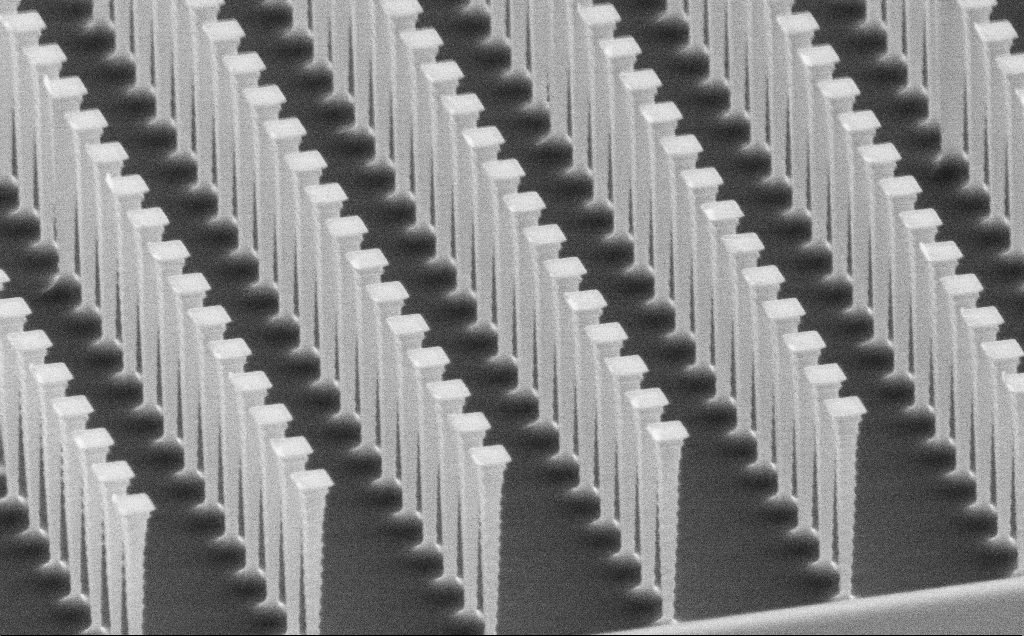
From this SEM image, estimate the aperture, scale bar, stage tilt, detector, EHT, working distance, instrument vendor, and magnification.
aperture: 30 µm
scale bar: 1000 nm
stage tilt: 62°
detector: SE2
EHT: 10 kV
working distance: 8 mm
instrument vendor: Zeiss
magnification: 13.67 K X